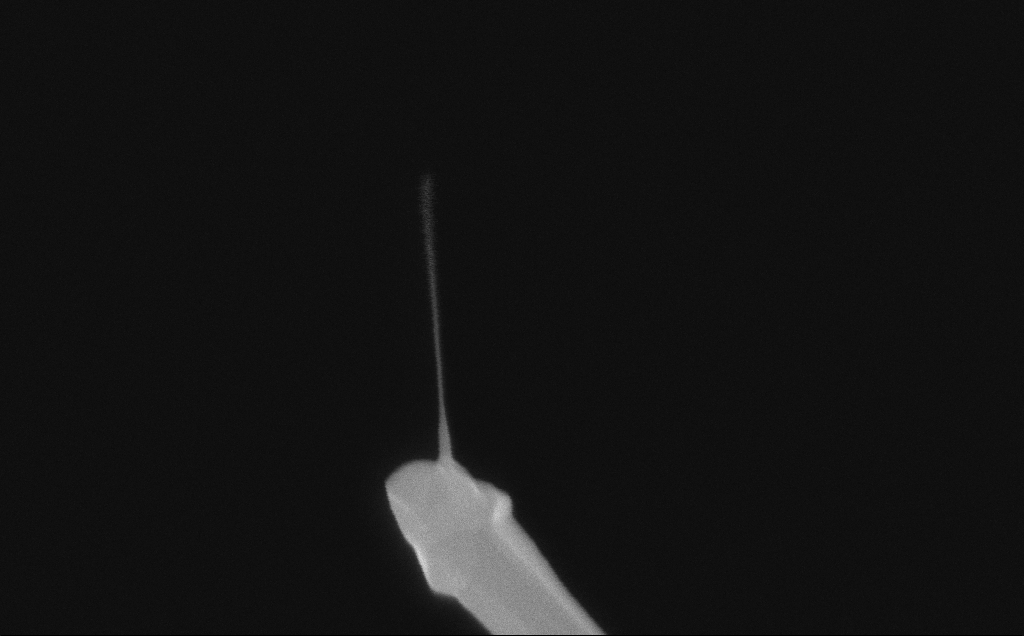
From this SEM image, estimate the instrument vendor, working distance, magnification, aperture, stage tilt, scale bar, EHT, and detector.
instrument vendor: Zeiss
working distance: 6 mm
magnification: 189.64 K X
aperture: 30 µm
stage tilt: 0°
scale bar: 200 nm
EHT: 10 kV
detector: InLens